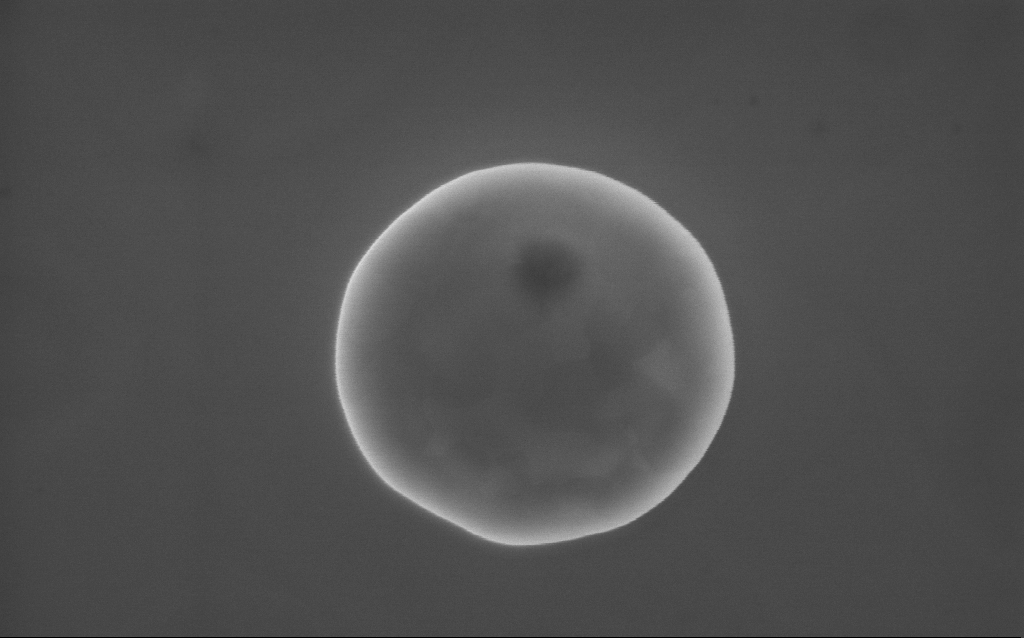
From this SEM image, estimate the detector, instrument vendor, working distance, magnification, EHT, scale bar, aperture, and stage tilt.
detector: InLens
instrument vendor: Zeiss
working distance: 2 mm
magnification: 115 K X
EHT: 10 kV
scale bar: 200 nm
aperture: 30 µm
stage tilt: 0°